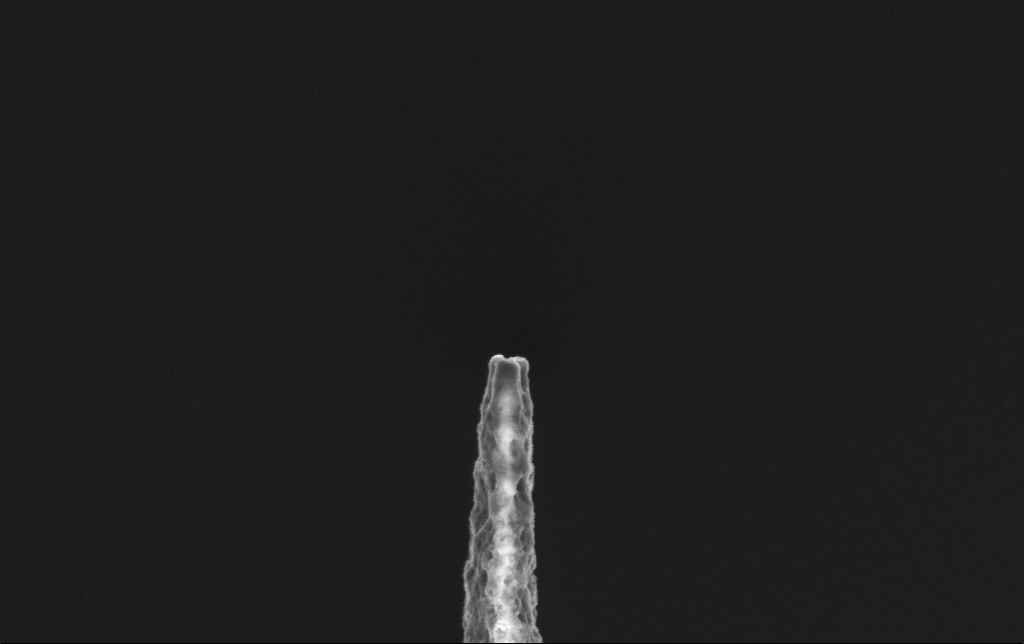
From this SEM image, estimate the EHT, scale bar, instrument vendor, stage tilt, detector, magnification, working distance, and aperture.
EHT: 2 kV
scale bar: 200 nm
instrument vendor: Zeiss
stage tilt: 0°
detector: InLens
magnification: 75 K X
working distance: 6.5 mm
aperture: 30 µm